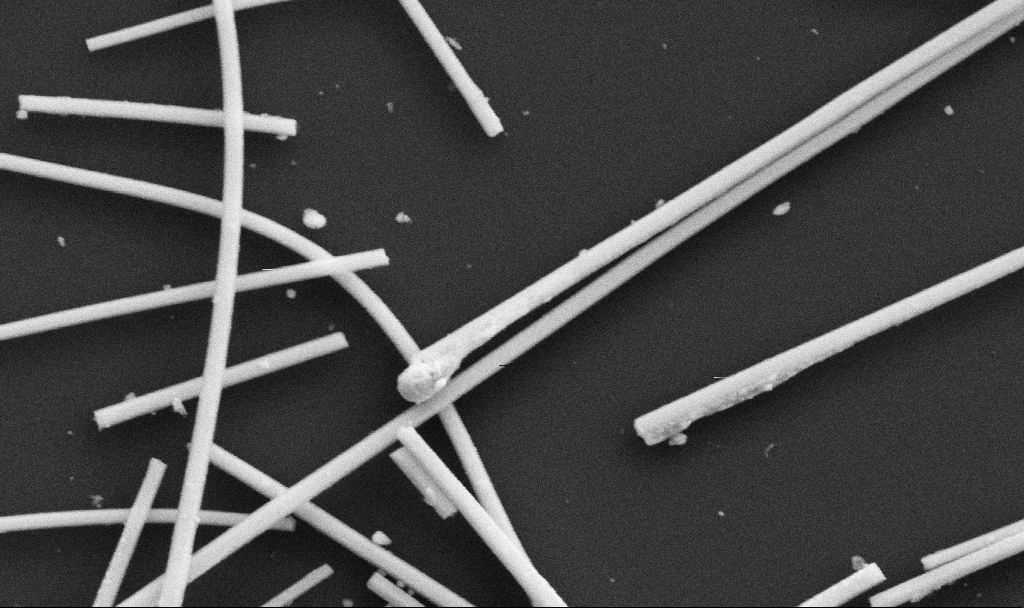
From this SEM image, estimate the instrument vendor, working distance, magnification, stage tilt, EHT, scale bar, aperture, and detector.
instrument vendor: Zeiss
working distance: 10.7 mm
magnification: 66.35 K X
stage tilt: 0°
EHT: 5 kV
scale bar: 1000 nm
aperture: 30 µm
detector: SE2